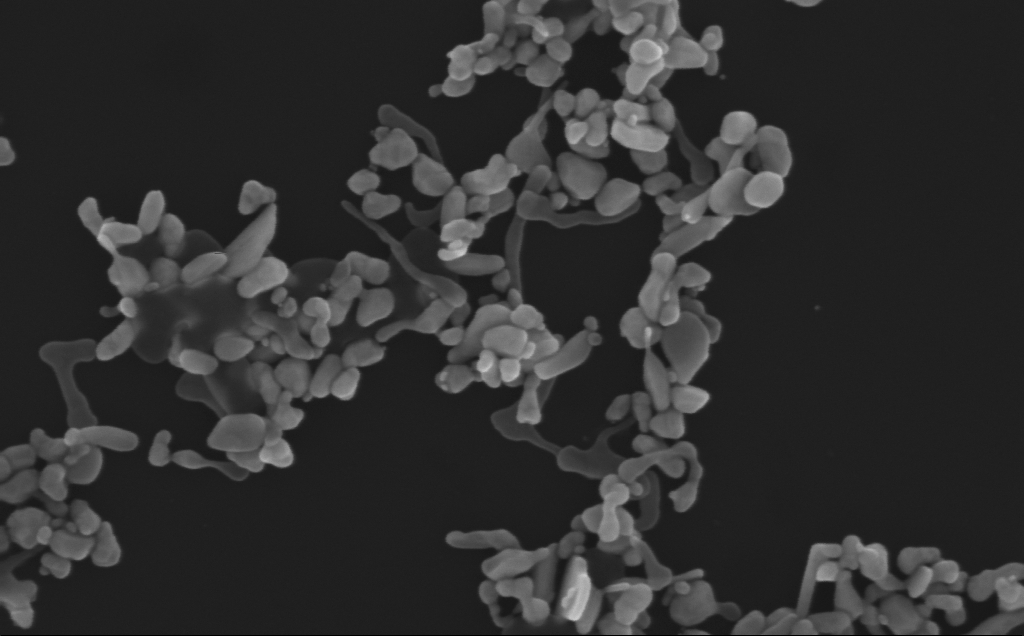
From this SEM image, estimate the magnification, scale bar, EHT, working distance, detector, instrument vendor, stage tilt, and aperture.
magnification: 186.07 K X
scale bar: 200 nm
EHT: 10 kV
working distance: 3 mm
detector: InLens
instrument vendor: Zeiss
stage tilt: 0°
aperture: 30 µm